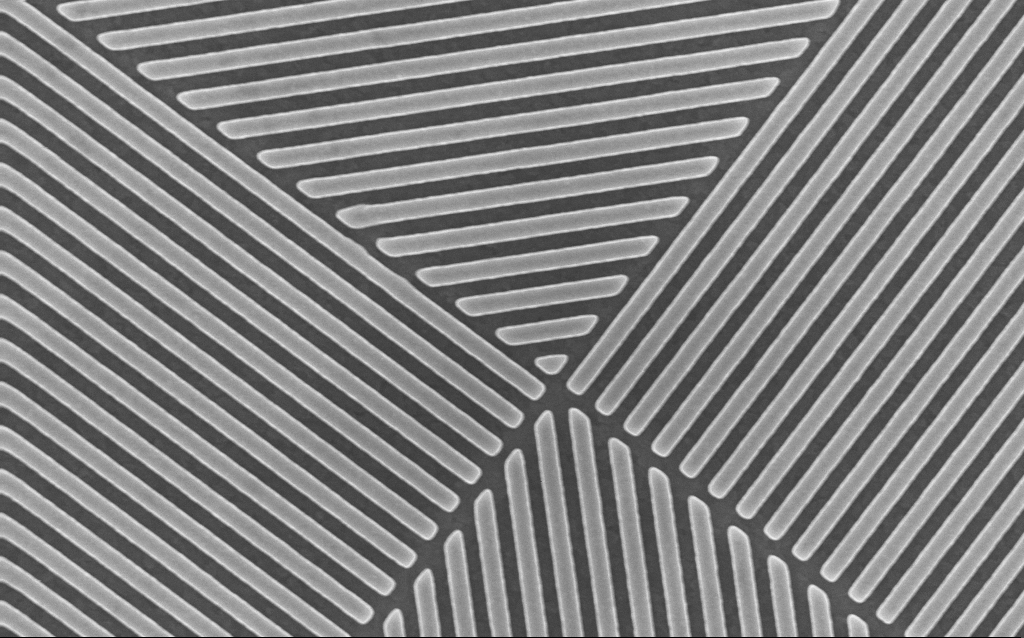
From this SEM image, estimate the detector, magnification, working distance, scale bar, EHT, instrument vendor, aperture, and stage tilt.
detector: InLens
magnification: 32.48 K X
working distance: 3.9 mm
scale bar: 2000 nm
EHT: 10 kV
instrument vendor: Zeiss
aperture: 30 µm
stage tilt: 0°